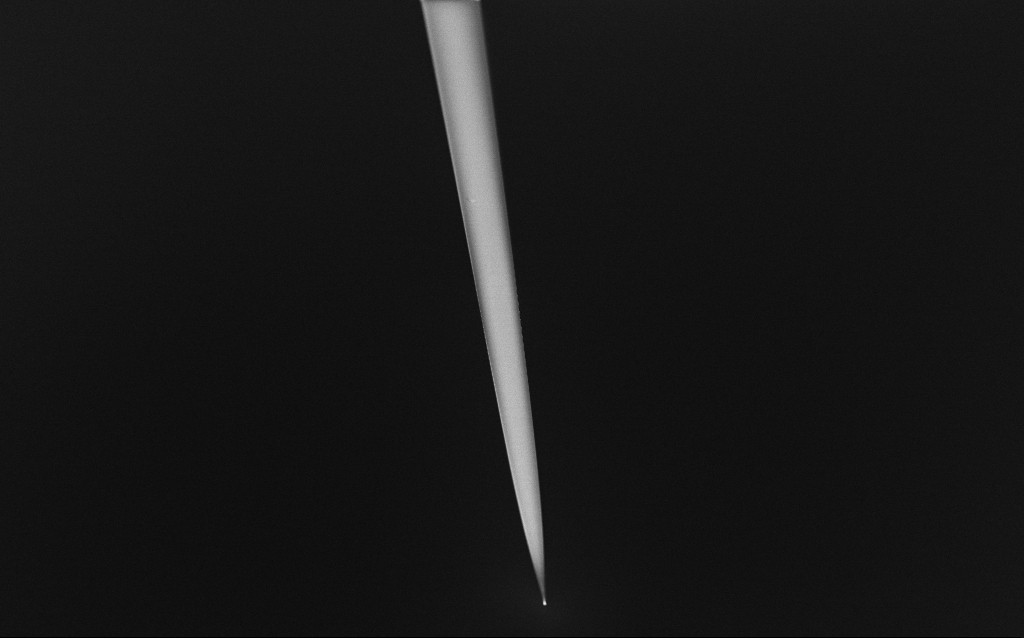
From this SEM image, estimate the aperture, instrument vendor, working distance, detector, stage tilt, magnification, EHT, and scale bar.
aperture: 30 µm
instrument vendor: Zeiss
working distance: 6 mm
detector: InLens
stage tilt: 45°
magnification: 0.5 K X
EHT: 1 kV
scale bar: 100000 nm